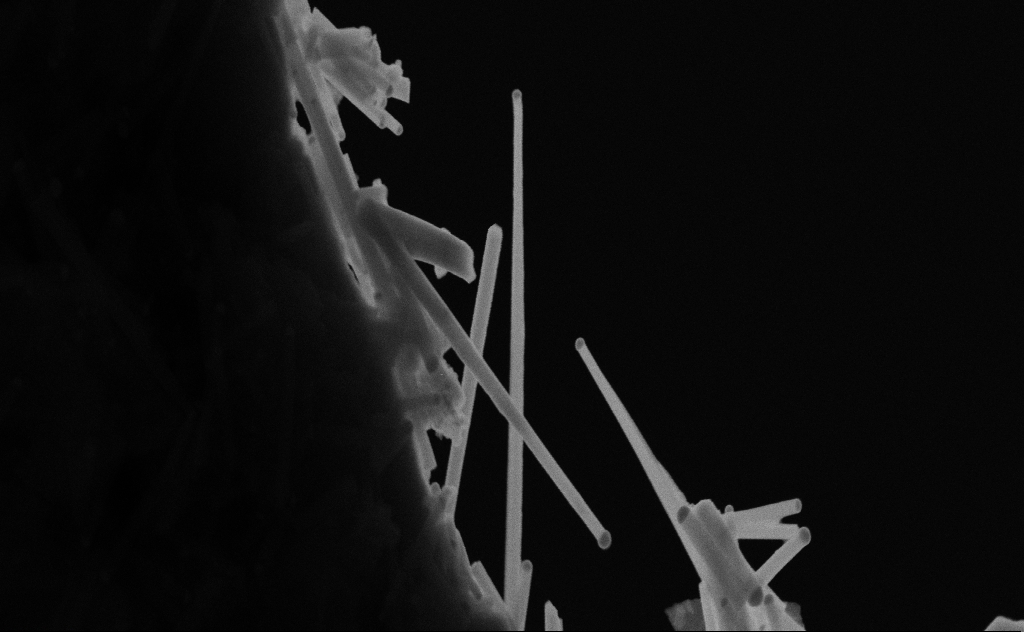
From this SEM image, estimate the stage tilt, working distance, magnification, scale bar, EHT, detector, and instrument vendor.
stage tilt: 0°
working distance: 9 mm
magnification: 72.53 K X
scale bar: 200 nm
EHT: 20 kV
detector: SE2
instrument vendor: Zeiss